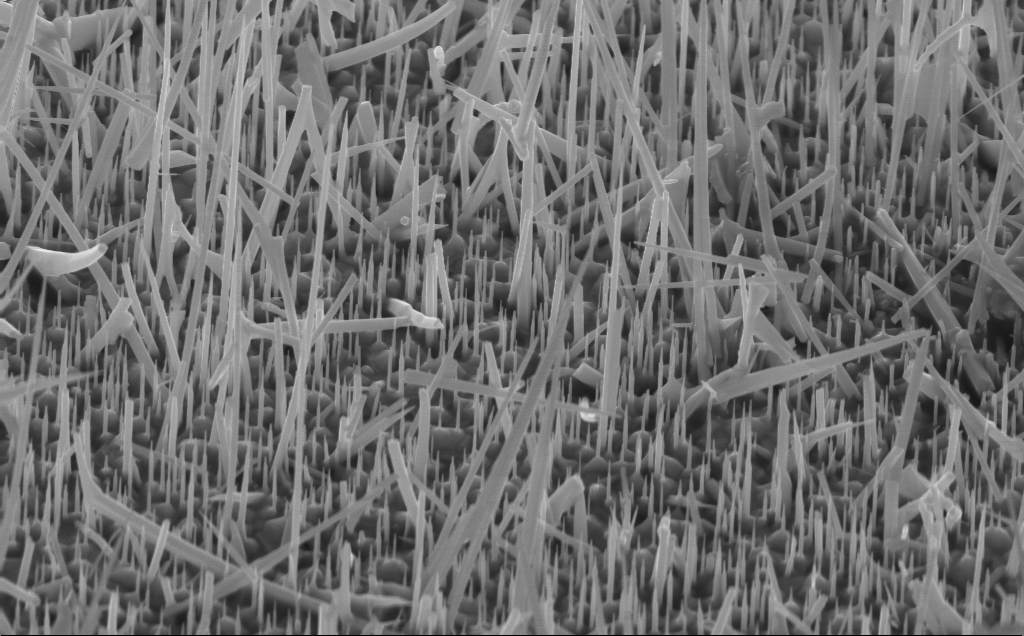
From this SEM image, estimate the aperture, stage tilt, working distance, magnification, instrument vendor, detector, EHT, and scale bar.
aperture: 30 µm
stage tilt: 45°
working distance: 4 mm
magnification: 22.66 K X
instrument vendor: Zeiss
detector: InLens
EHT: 10 kV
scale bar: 2000 nm